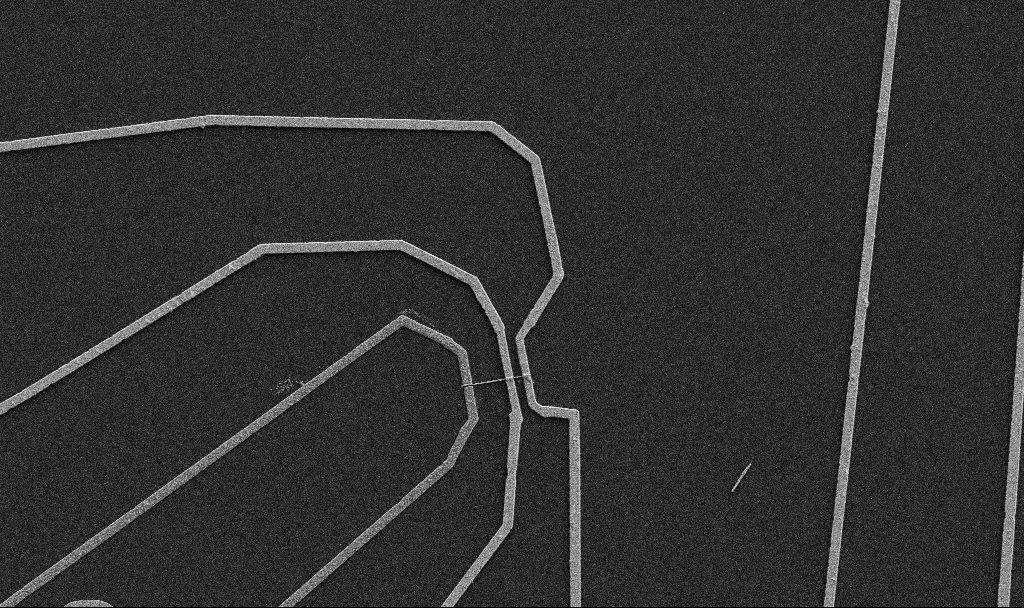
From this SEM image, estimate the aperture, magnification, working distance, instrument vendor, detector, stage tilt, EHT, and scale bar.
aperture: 30 µm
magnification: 5 K X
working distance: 10.7 mm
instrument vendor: Zeiss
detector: SE2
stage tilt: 0°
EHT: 5 kV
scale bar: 10000 nm